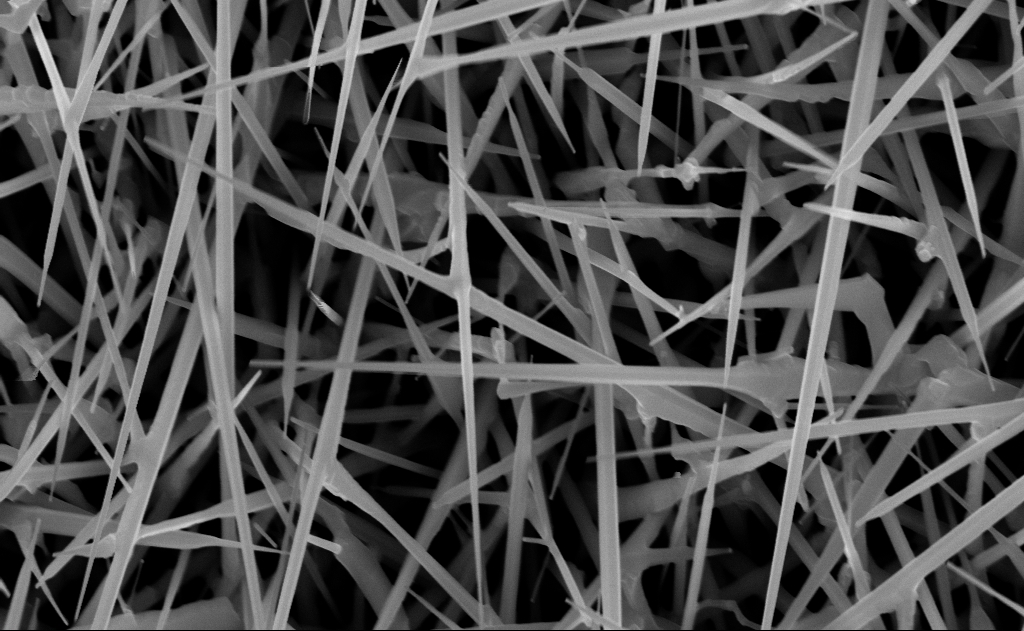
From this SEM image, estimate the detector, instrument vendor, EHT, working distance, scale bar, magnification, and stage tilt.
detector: InLens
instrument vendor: Zeiss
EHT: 10 kV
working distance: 10 mm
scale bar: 1000 nm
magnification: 40 K X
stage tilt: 0°